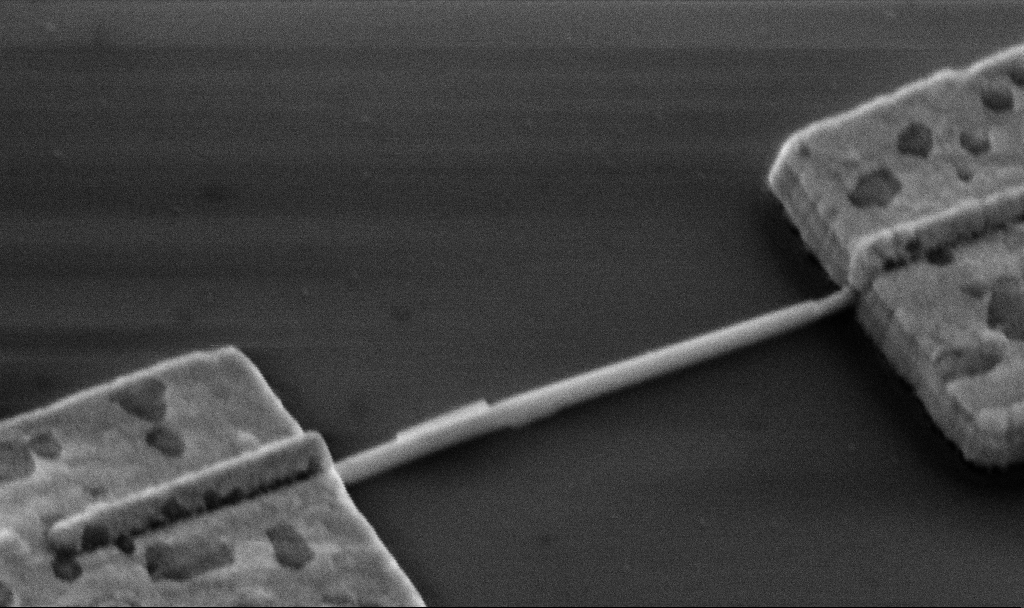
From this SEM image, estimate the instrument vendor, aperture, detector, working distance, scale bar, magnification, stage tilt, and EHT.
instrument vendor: Zeiss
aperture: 30 µm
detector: SE2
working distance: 13.6 mm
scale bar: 200 nm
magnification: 80.17 K X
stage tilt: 45°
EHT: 5 kV